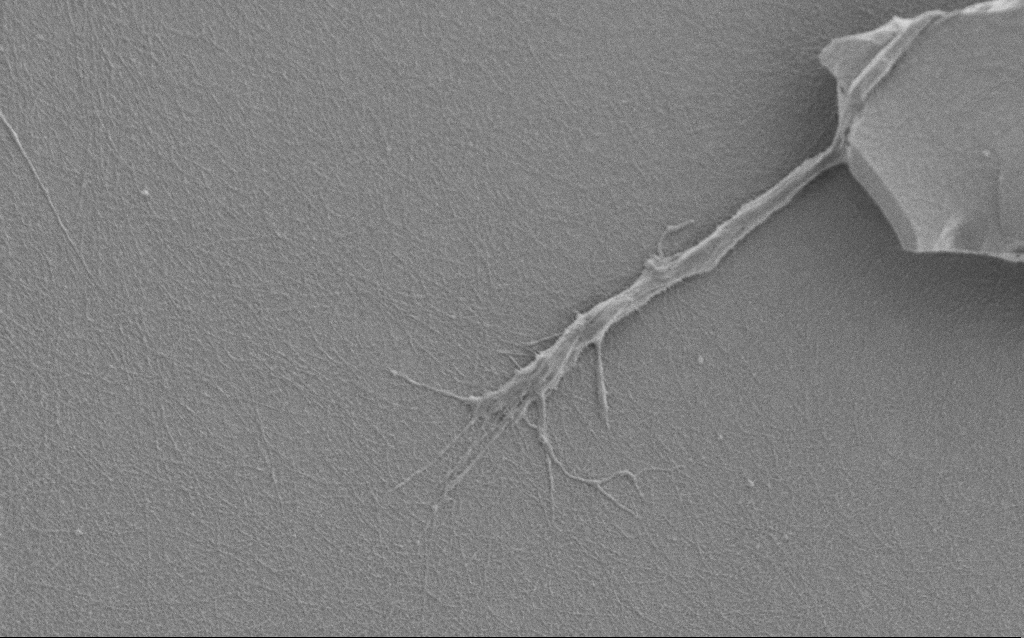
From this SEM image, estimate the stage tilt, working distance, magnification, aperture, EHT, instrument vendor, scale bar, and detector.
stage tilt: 0°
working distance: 6 mm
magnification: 7.5 K X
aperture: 30 µm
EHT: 1 kV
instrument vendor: Zeiss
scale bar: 2000 nm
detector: SE2